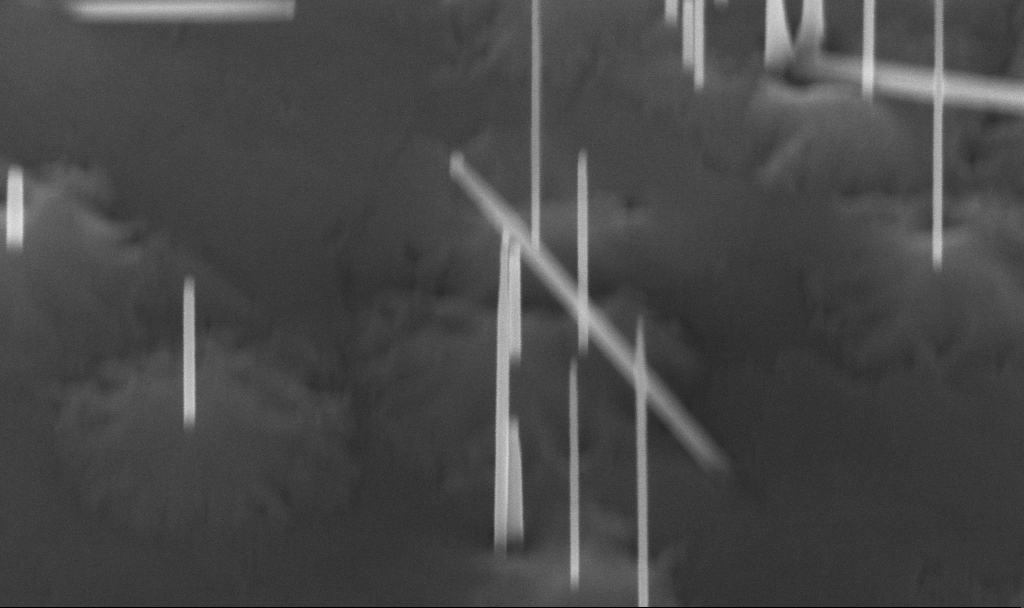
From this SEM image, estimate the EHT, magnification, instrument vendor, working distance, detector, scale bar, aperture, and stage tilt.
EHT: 10 kV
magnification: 155.88 K X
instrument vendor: Zeiss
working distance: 5.6 mm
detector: InLens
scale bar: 200 nm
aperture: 30 µm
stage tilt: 45°